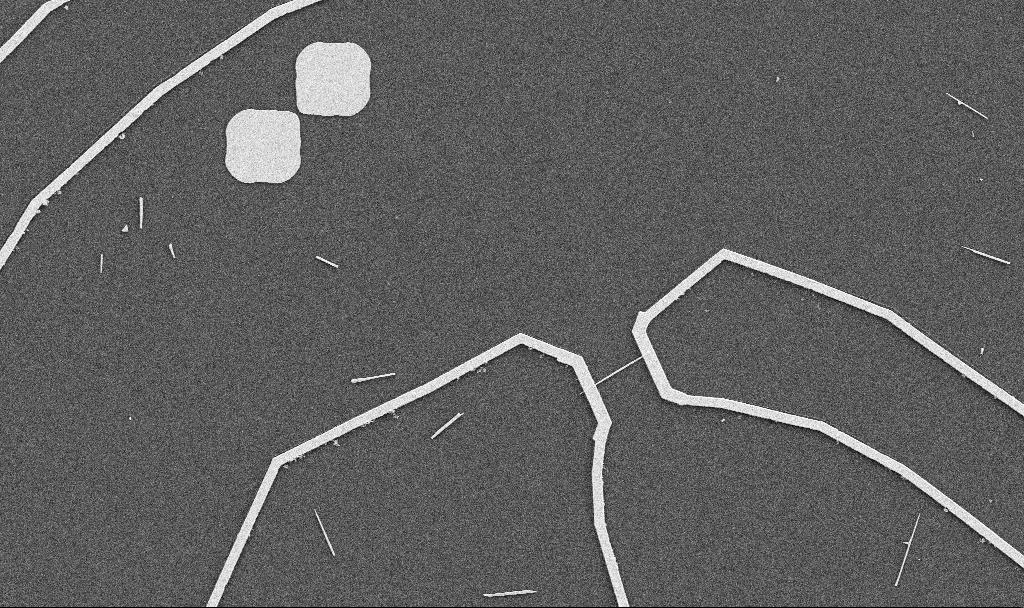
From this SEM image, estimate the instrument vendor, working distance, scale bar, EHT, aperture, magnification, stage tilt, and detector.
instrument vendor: Zeiss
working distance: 10.7 mm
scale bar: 10000 nm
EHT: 5 kV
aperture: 30 µm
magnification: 5 K X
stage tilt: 0°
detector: SE2